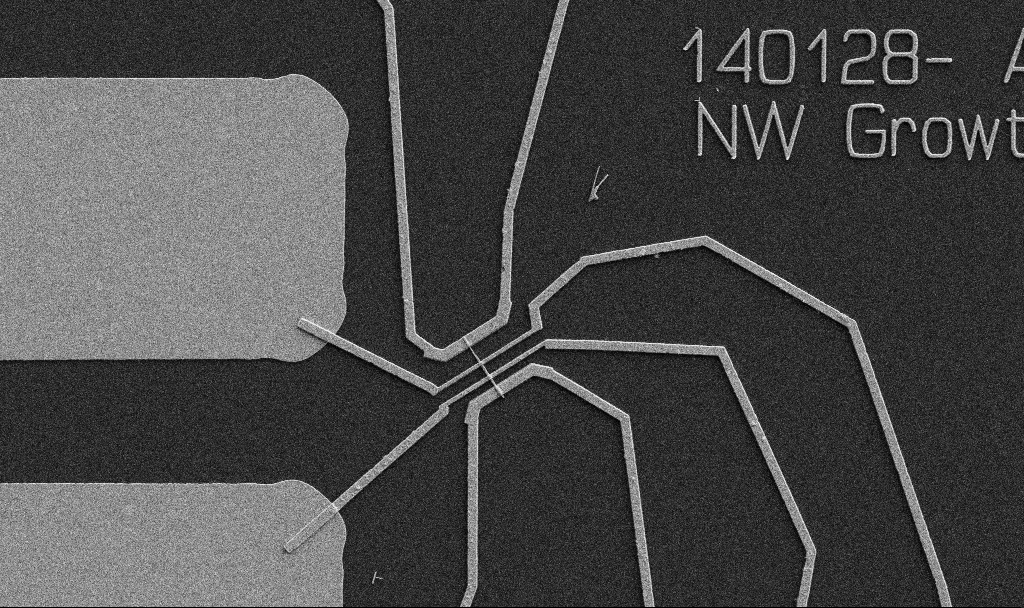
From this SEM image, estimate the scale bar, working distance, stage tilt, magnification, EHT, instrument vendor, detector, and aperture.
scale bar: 10000 nm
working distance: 10.7 mm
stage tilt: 0°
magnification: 5 K X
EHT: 5 kV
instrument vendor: Zeiss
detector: SE2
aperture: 30 µm